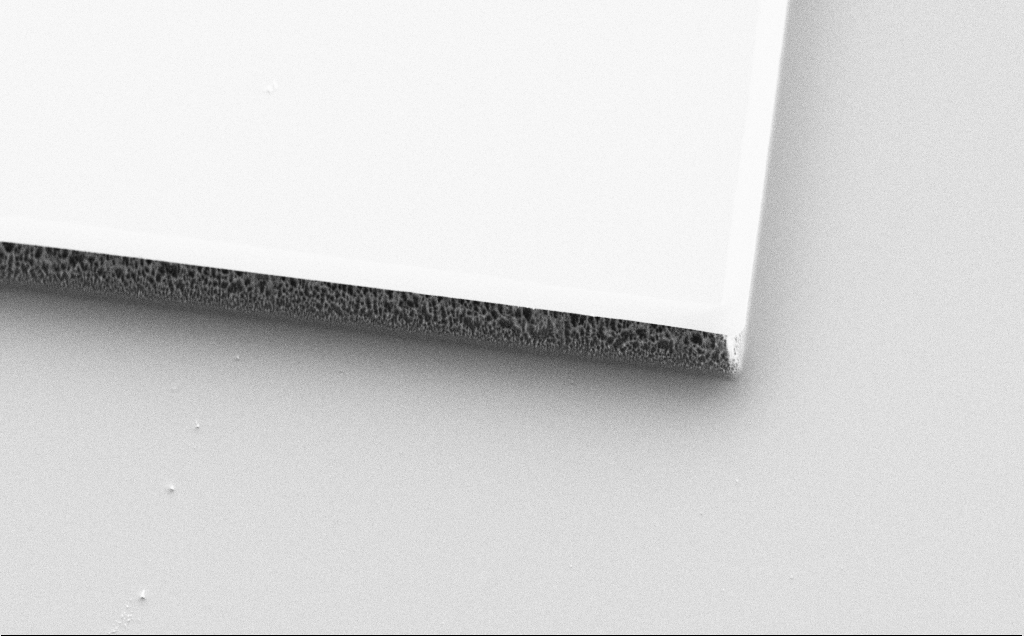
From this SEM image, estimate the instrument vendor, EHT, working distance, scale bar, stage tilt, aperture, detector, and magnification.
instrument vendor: Zeiss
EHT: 5 kV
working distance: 7 mm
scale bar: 20000 nm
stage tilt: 45°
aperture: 30 µm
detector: SE2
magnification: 1.5 K X